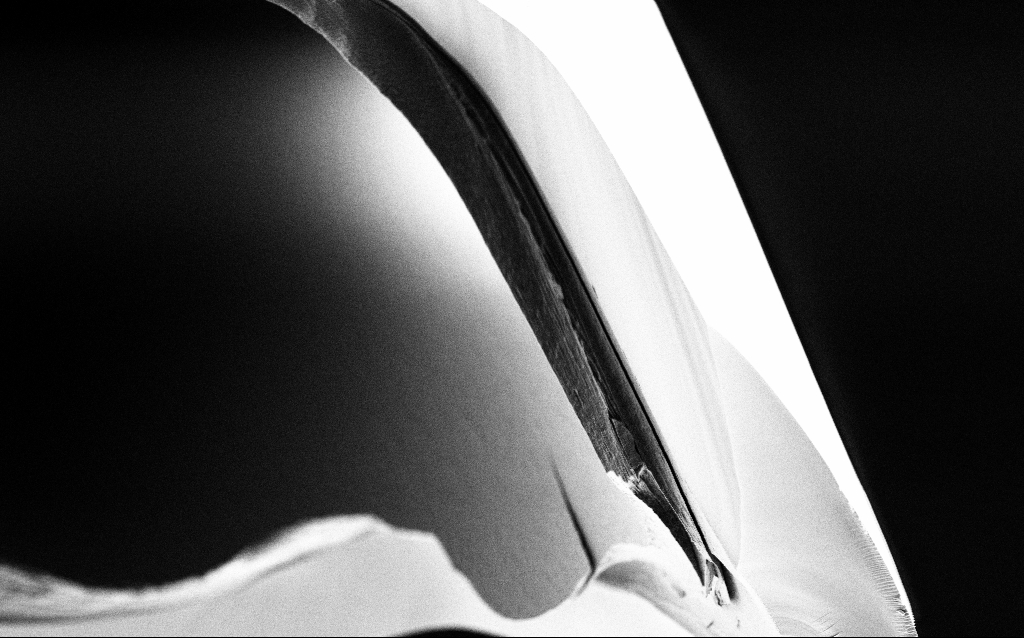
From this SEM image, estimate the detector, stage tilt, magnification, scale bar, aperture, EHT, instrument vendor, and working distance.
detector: SE2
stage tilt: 45°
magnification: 10 K X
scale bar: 2000 nm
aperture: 30 µm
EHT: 1 kV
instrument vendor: Zeiss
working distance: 6.4 mm